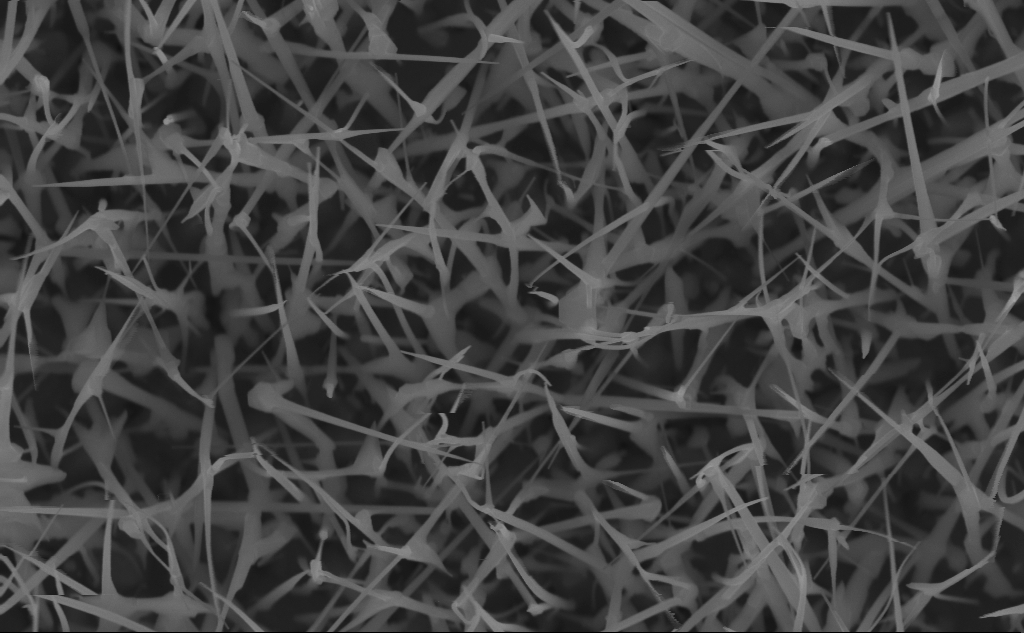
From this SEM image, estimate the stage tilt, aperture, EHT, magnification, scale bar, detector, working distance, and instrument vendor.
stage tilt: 0°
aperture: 30 µm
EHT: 10 kV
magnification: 58.56 K X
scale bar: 1000 nm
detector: InLens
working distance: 6 mm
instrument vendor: Zeiss